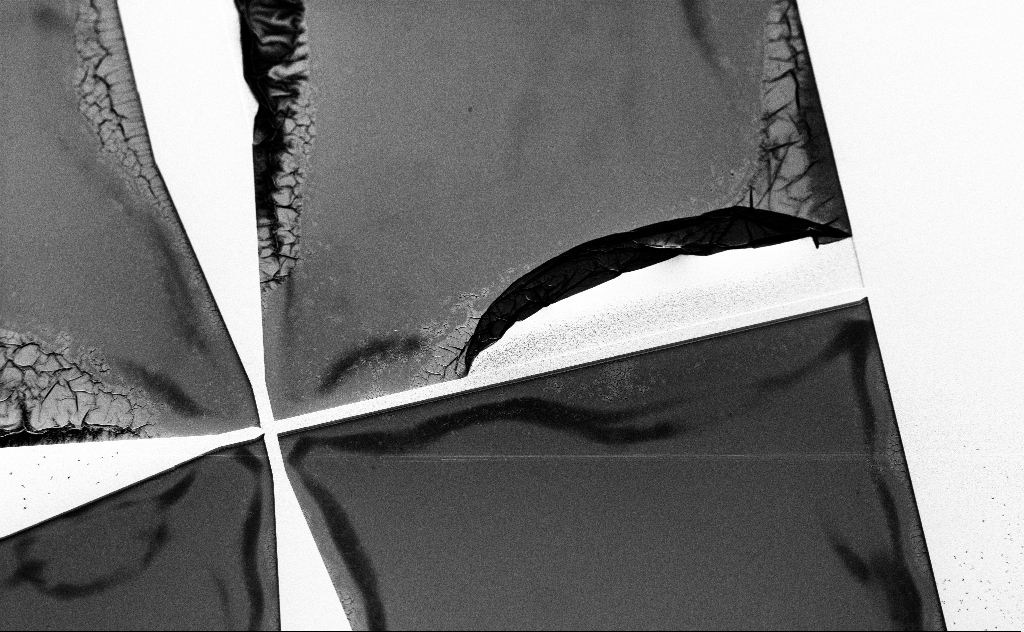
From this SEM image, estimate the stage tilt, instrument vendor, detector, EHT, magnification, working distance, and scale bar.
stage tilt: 45°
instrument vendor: Zeiss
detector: SE2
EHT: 3 kV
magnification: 0.442 K X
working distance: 11 mm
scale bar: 100000 nm